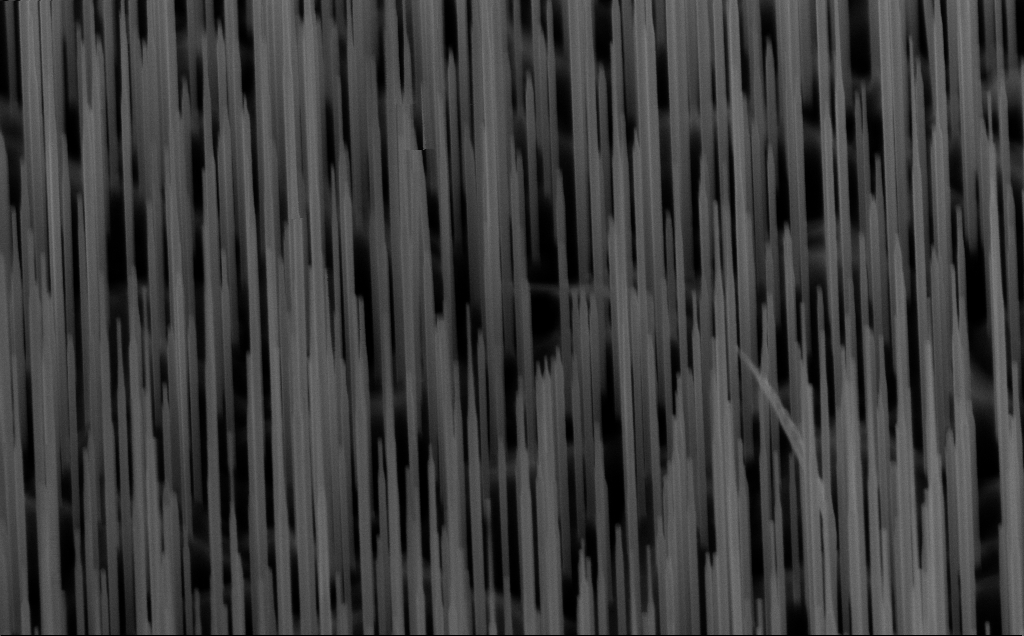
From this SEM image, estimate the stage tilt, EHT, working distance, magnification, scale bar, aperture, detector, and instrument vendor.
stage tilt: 45°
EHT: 10 kV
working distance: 6 mm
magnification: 40 K X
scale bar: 1000 nm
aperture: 30 µm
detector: InLens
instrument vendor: Zeiss